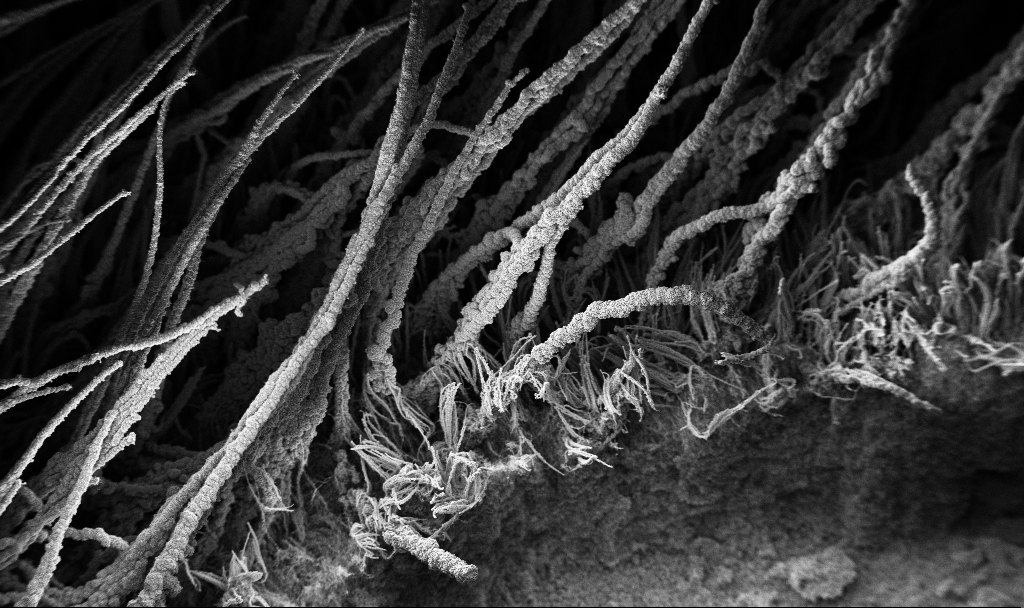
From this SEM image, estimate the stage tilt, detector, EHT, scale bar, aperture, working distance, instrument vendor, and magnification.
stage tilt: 37.7°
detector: InLens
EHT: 3 kV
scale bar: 100000 nm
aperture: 30 µm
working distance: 7.5 mm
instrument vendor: Zeiss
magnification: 0.15 K X